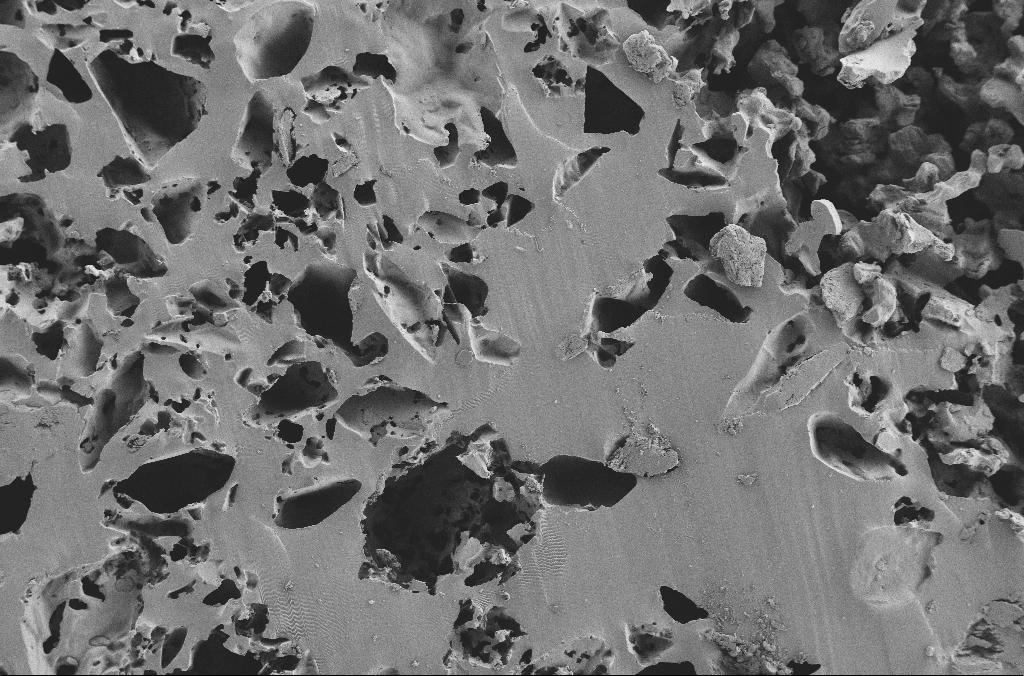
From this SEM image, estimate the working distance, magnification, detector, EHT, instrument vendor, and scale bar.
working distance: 3.1 mm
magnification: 0.25 K X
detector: SE2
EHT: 2 kV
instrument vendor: Zeiss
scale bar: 100000 nm